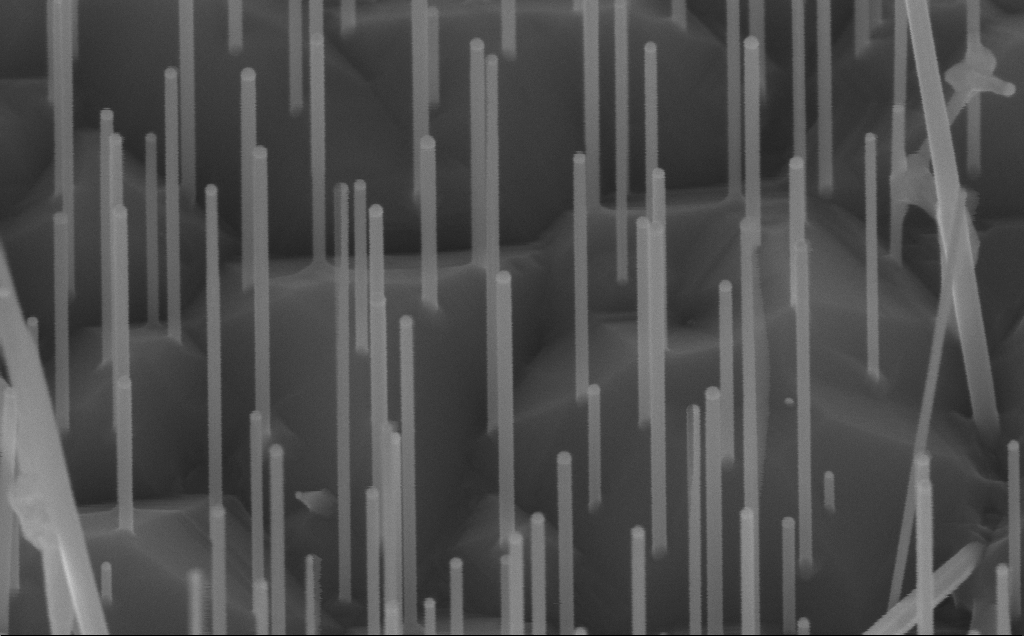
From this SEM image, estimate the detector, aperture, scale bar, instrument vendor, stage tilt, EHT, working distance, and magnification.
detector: InLens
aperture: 30 µm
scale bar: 200 nm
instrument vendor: Zeiss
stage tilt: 45°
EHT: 10 kV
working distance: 8 mm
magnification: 154.26 K X